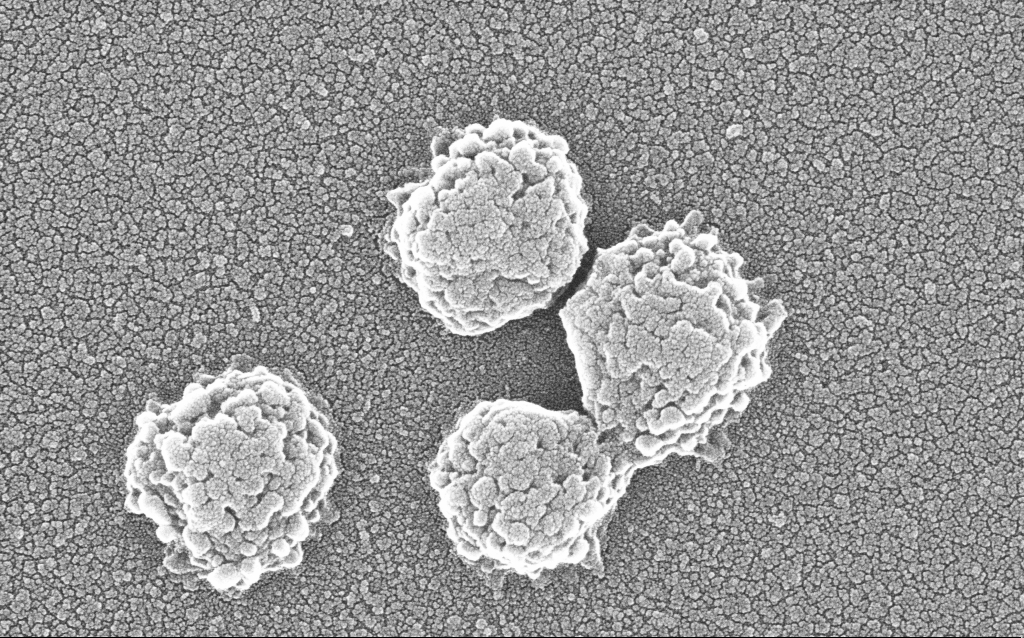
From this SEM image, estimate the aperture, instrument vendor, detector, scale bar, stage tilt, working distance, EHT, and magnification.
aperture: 30 µm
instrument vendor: Zeiss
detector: InLens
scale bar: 200 nm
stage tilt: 0°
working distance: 1.8 mm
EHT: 20 kV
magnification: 200 K X